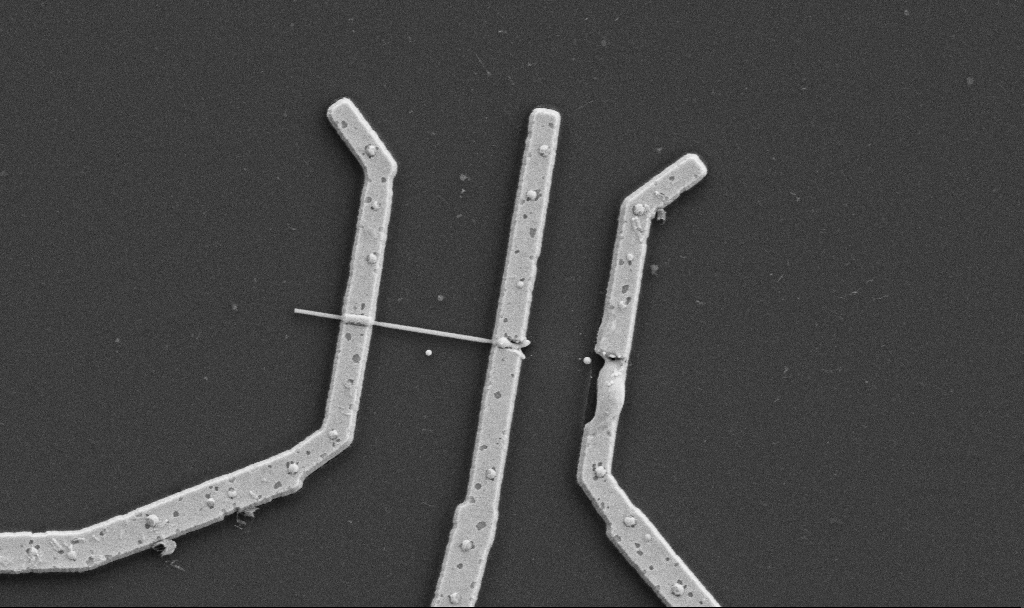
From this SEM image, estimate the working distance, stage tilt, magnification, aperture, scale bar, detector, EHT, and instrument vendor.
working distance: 10.6 mm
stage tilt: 0°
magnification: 20 K X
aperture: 30 µm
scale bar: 1000 nm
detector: SE2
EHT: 5 kV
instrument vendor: Zeiss